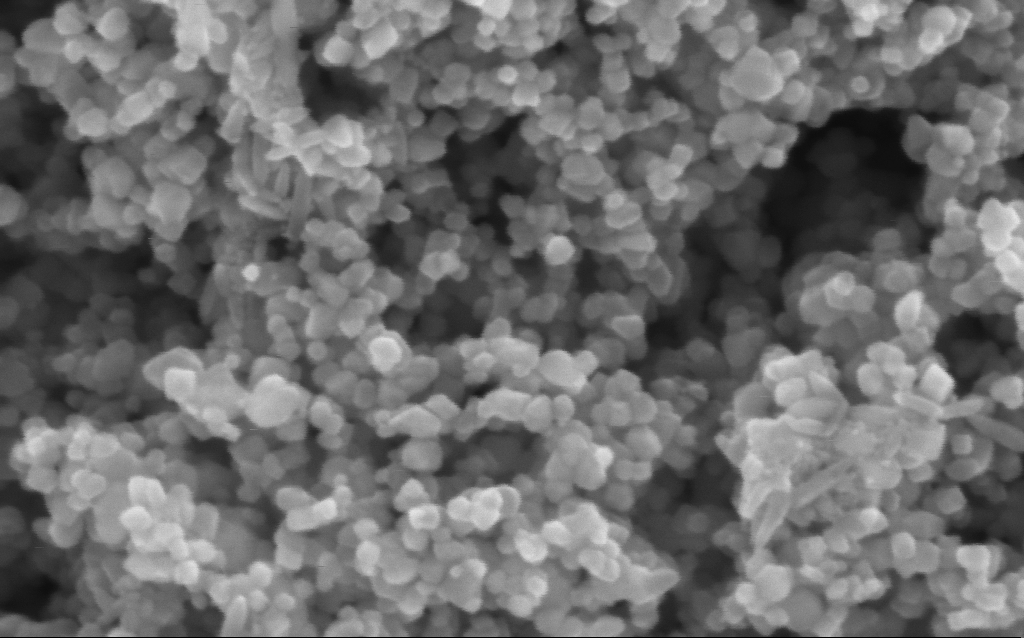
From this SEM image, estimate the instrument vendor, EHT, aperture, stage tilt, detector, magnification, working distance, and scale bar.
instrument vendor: Zeiss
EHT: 5 kV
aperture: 30 µm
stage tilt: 0°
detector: InLens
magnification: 416 K X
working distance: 4.4 mm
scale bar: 100 nm